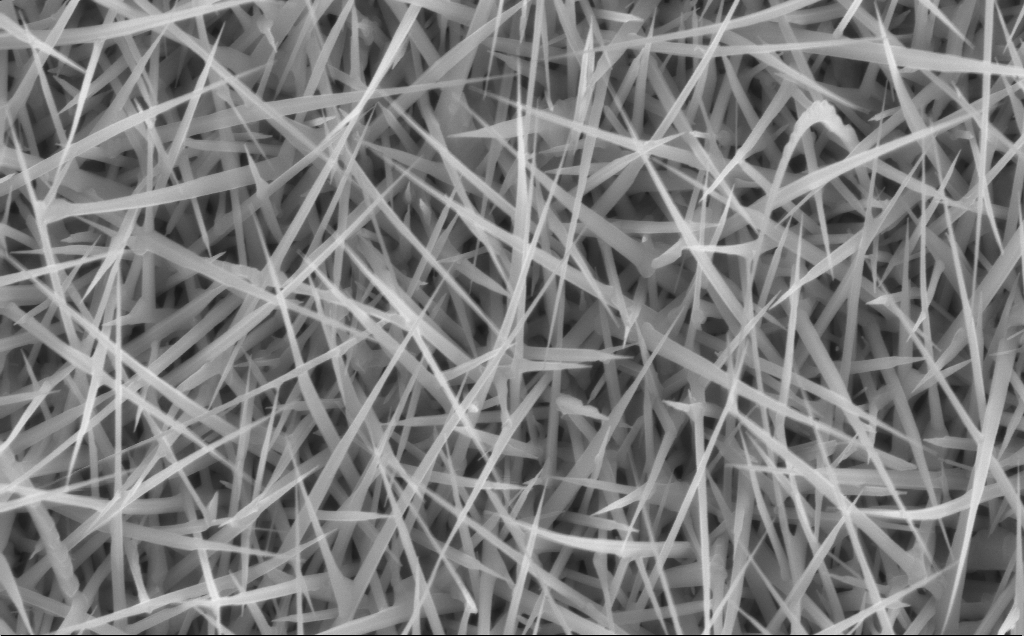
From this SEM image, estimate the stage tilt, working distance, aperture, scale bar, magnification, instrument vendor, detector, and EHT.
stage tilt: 0°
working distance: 7 mm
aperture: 30 µm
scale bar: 1000 nm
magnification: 40 K X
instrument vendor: Zeiss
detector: InLens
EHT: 10 kV